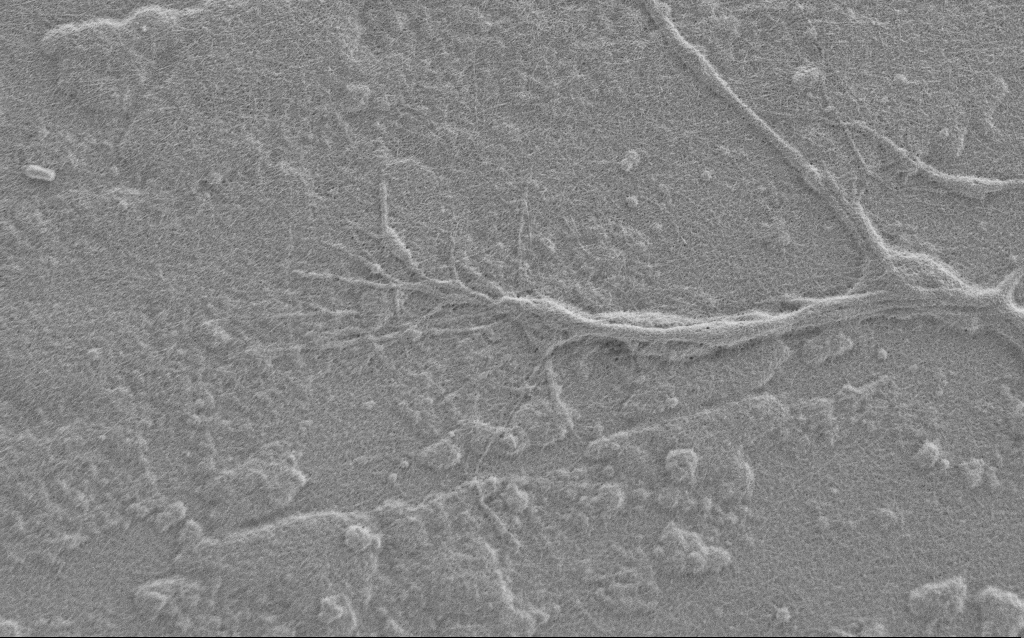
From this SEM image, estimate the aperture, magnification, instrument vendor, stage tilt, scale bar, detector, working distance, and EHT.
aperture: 30 µm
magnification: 7.5 K X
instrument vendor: Zeiss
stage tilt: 0°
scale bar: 2000 nm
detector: SE2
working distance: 6 mm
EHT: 1 kV